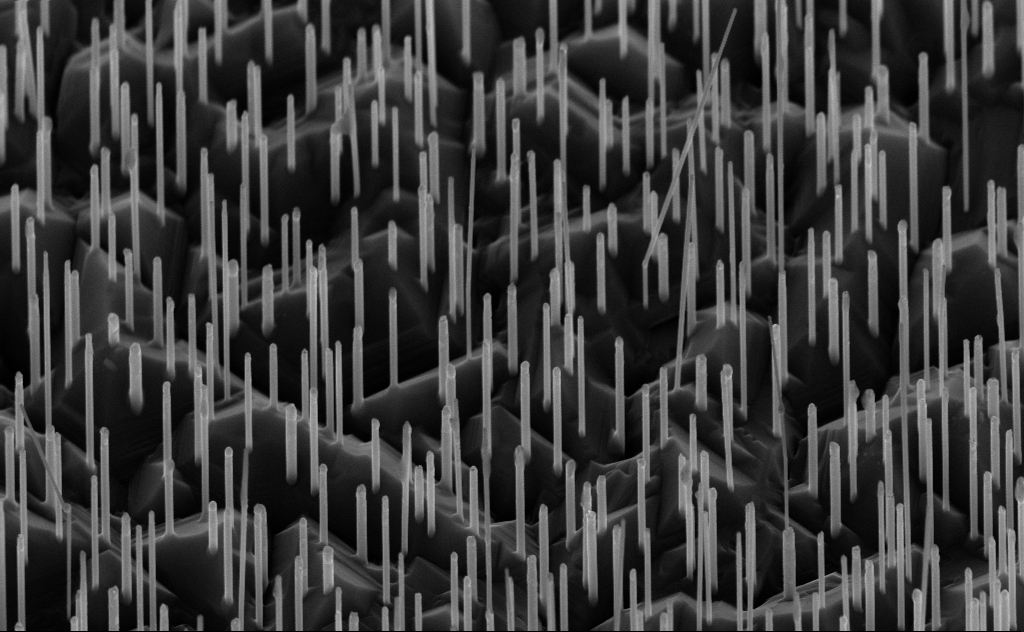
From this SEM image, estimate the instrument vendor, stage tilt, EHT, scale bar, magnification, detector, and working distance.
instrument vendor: Zeiss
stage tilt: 45°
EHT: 10 kV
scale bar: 1000 nm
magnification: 40 K X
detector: InLens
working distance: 6 mm